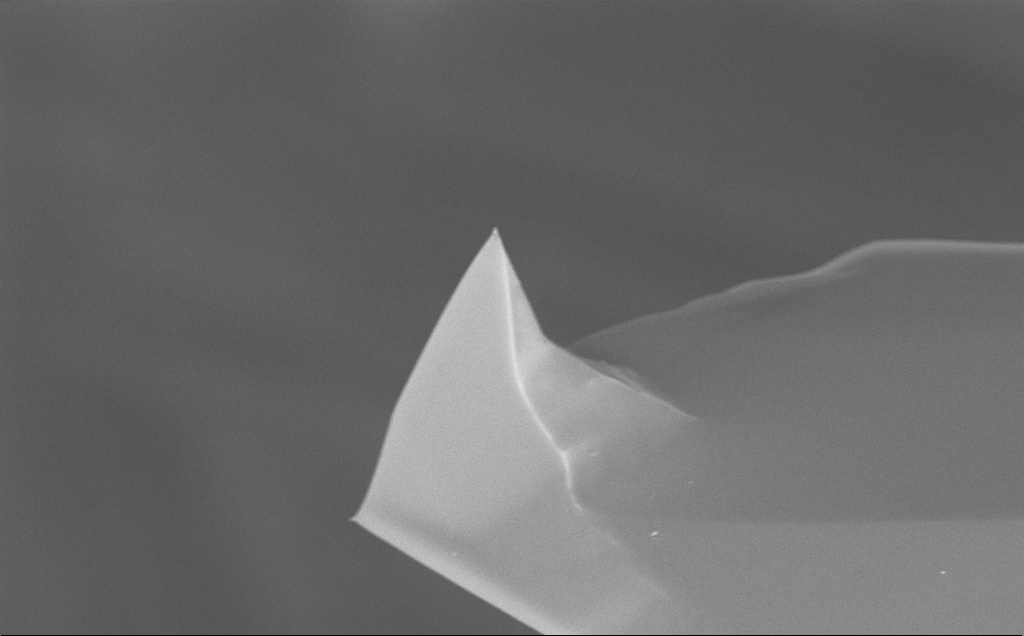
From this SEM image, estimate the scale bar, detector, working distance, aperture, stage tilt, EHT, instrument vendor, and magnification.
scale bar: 2000 nm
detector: InLens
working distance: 14 mm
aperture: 30 µm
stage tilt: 52.7°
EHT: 10 kV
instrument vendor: Zeiss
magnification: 7.8 K X